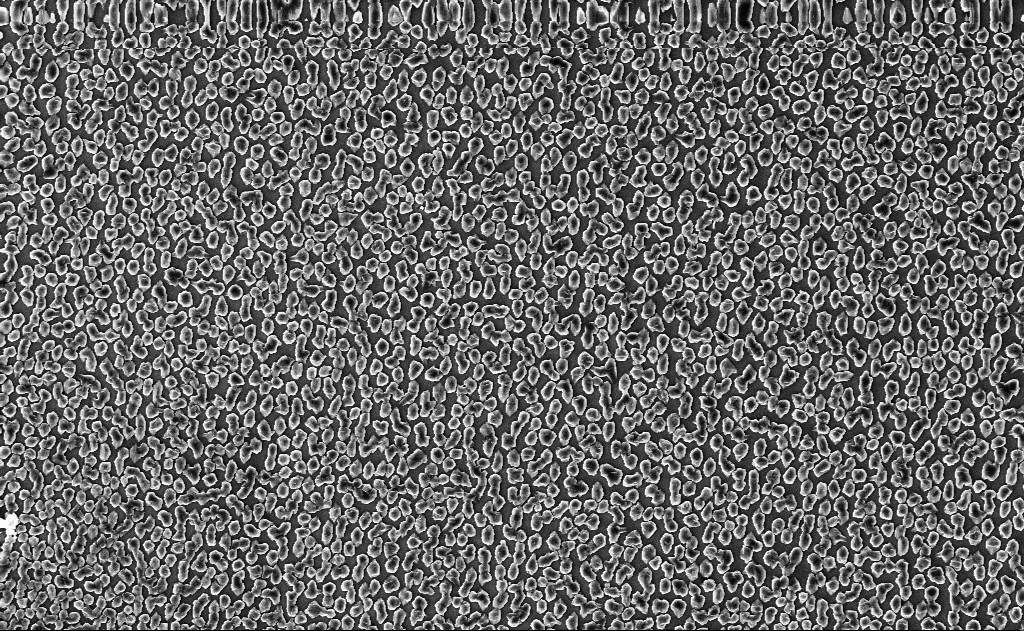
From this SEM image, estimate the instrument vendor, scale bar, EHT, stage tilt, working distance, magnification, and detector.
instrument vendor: Zeiss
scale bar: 10000 nm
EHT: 10 kV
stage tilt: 0°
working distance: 12 mm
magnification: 5 K X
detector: InLens